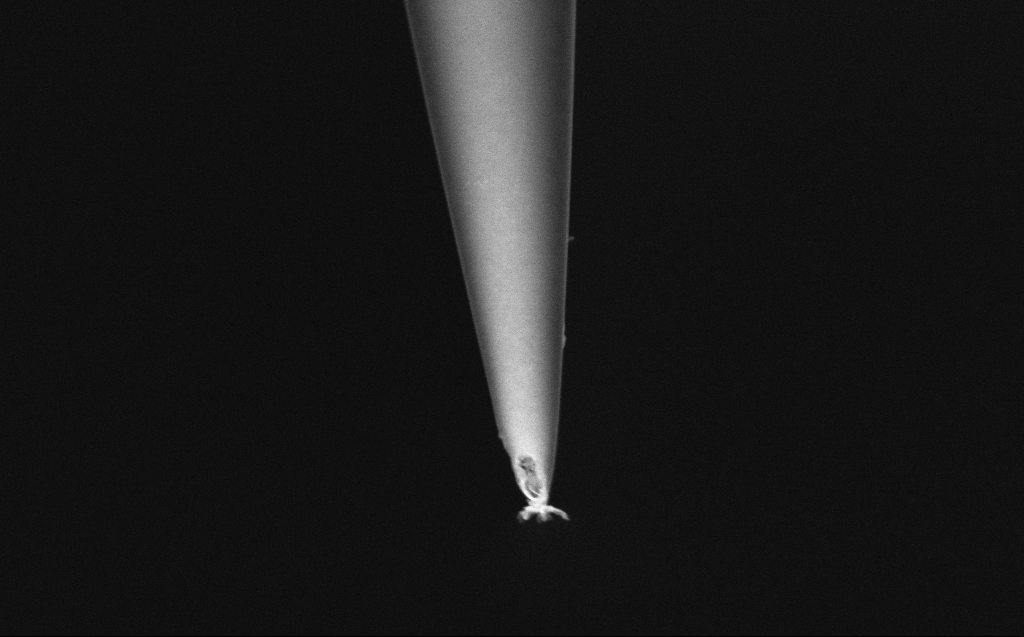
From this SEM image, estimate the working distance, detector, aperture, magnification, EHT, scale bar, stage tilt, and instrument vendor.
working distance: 6 mm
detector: InLens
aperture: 30 µm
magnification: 50 K X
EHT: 2 kV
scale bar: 1000 nm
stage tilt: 45°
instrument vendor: Zeiss